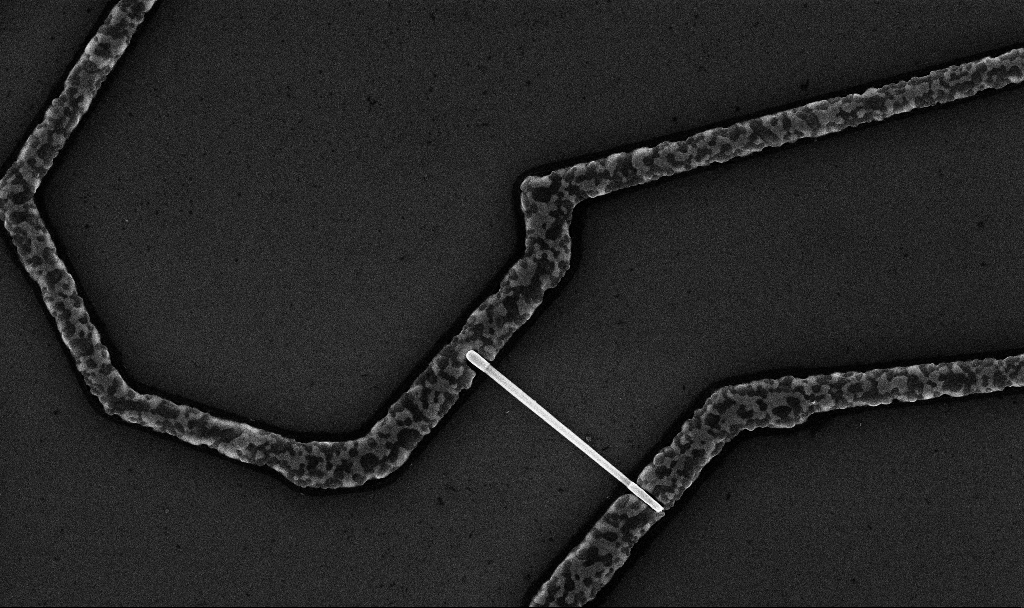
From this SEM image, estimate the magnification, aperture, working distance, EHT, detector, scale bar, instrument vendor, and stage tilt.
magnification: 20 K X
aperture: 30 µm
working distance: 6.7 mm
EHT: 10 kV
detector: InLens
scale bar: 1000 nm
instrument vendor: Zeiss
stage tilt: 0°